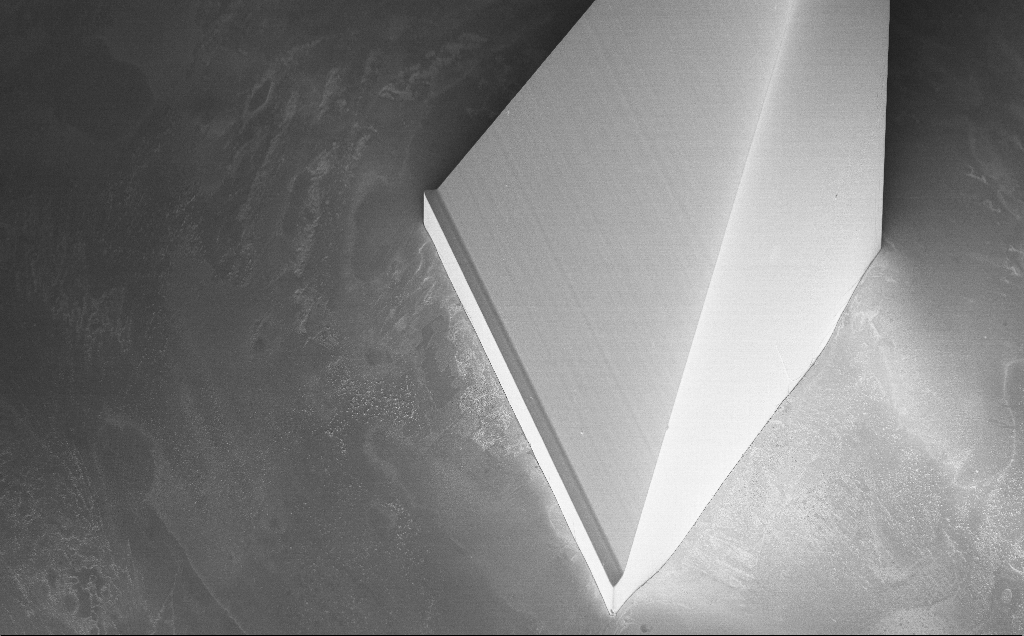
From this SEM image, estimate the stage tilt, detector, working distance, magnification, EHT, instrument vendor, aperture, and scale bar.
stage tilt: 40°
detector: InLens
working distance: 9 mm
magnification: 0.274 K X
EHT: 5 kV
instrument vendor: Zeiss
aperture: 30 µm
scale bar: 100000 nm